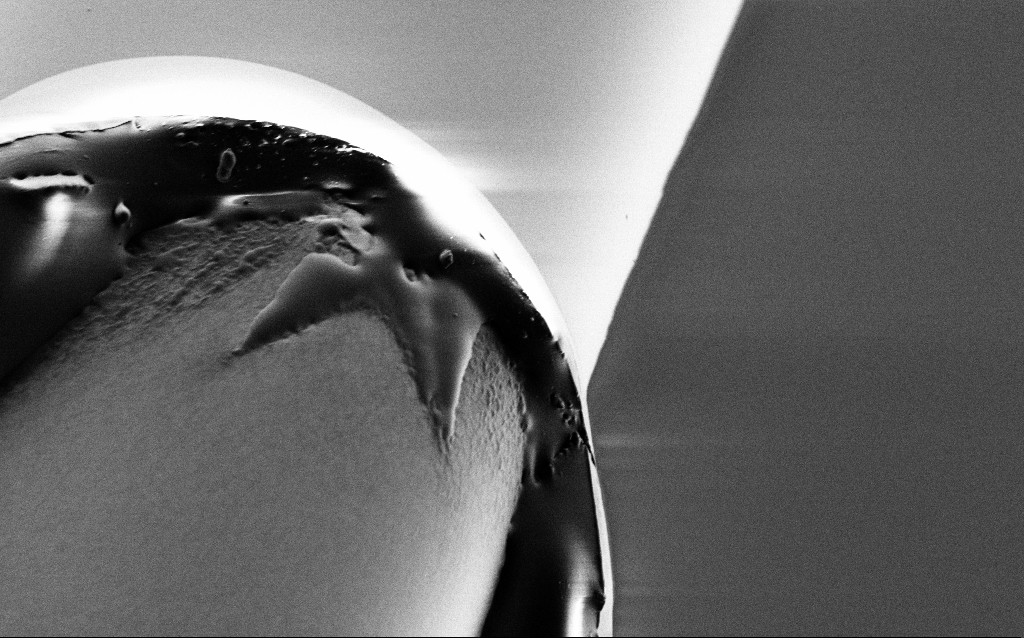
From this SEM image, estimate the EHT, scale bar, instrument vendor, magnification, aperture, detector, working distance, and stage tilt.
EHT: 1 kV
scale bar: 2000 nm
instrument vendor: Zeiss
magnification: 15 K X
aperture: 30 µm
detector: SE2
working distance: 6.1 mm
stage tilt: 45°